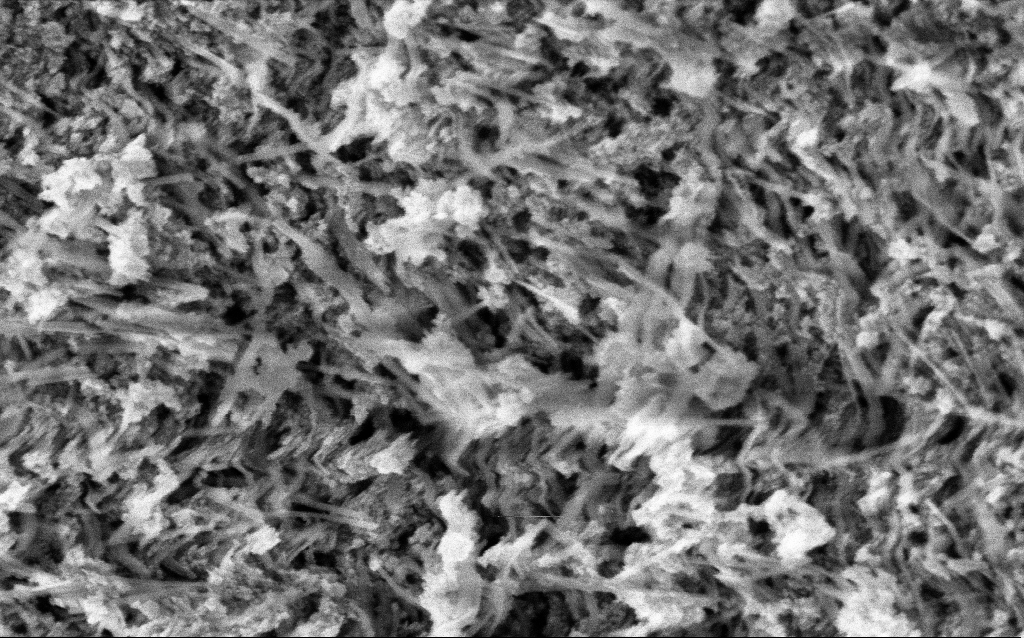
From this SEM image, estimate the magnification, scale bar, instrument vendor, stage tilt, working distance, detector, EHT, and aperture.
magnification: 166.69 K X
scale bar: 100 nm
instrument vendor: Zeiss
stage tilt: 0°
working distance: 5.1 mm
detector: InLens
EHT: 3 kV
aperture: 30 µm